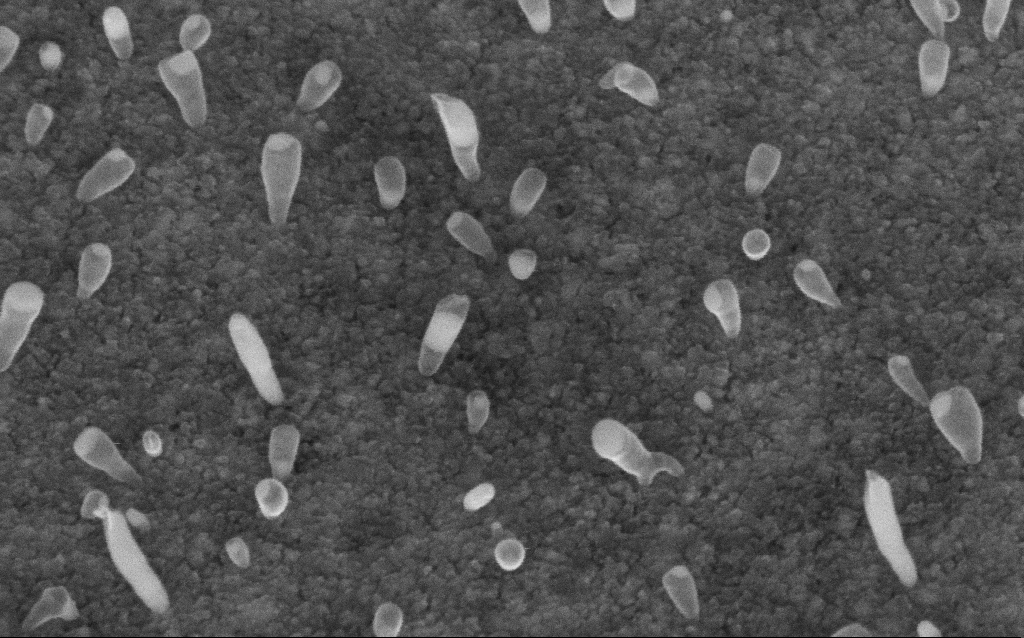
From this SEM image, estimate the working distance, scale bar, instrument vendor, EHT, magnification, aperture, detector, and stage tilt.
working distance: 7.2 mm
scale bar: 100 nm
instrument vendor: Zeiss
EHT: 5 kV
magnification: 200 K X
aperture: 30 µm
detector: InLens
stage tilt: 45°